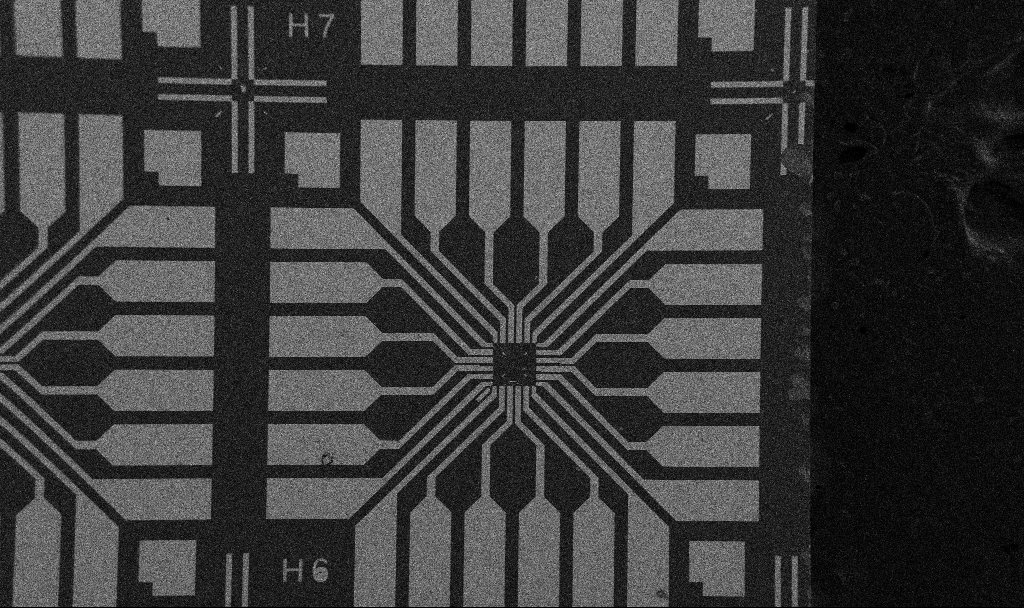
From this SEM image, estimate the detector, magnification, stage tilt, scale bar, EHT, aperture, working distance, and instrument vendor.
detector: SE2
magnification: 0.1 K X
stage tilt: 0°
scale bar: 200000 nm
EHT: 5 kV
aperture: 30 µm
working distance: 10.7 mm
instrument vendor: Zeiss